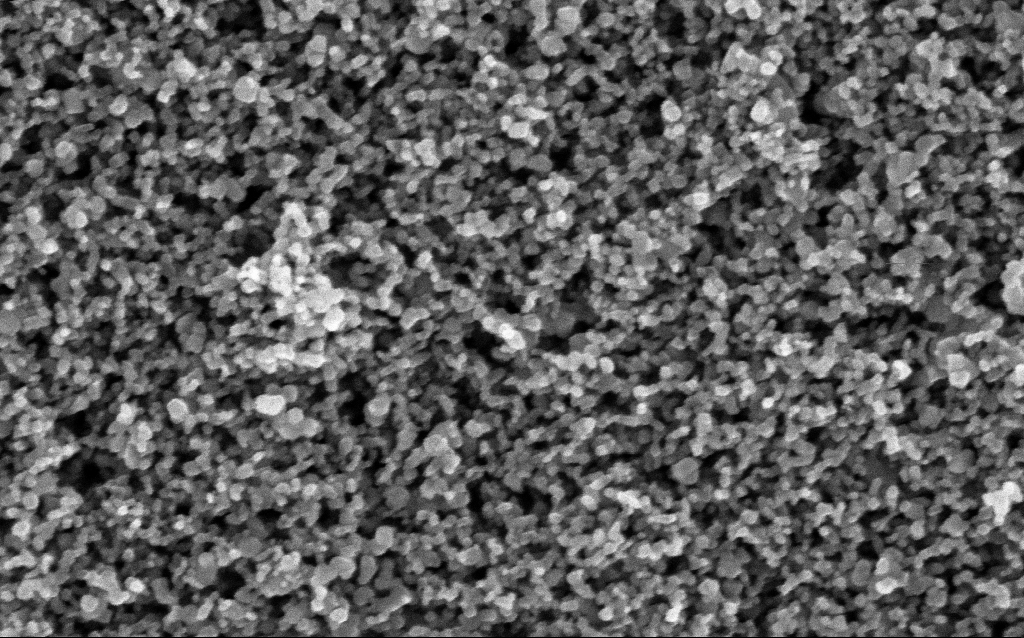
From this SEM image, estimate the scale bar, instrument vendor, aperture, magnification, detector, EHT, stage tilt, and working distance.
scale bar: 100 nm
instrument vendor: Zeiss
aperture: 30 µm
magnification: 162.39 K X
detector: InLens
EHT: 5 kV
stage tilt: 0°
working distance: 4.9 mm